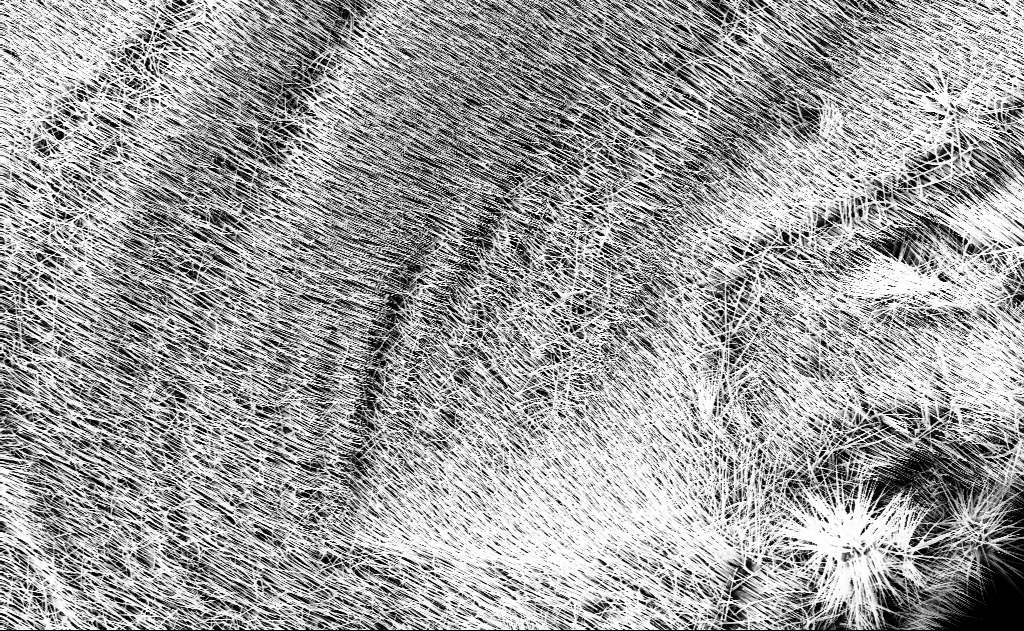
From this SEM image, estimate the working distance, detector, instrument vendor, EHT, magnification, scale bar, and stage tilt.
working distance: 13 mm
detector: InLens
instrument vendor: Zeiss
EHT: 10 kV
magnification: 5 K X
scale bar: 10000 nm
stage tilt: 0°